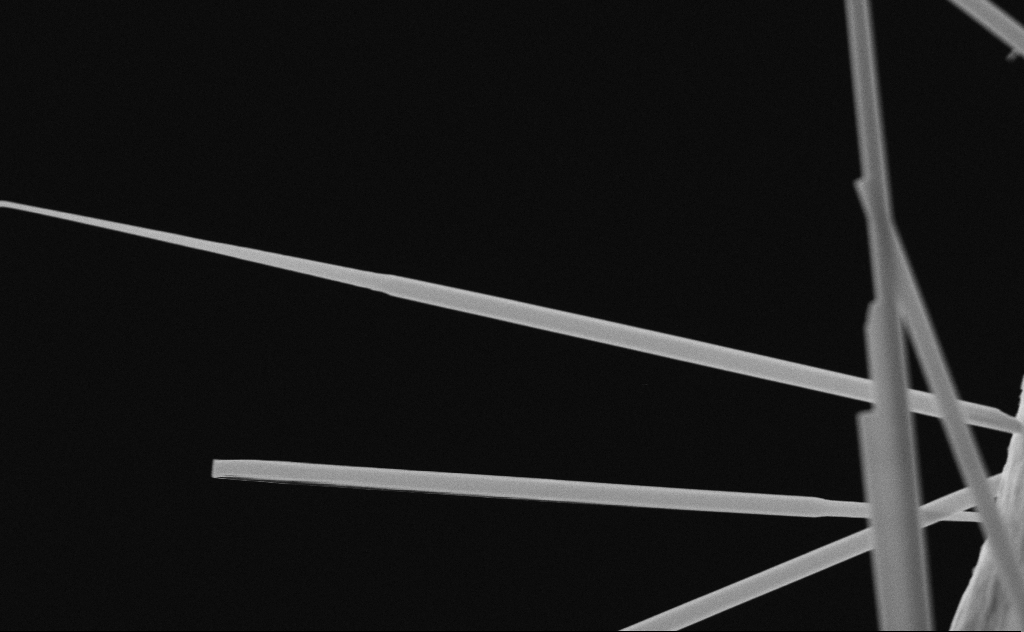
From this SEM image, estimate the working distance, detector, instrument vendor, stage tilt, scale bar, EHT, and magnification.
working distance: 10 mm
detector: SE2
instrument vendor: Zeiss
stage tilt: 0°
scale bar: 1000 nm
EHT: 20 kV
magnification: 55.11 K X